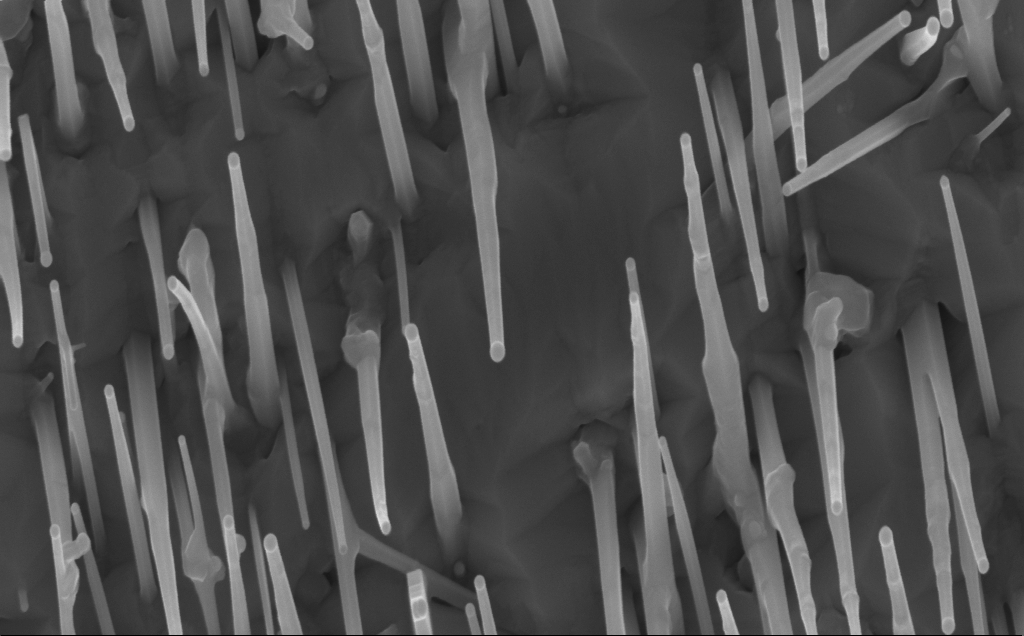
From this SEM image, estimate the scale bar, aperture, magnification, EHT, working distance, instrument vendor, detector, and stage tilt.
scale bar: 200 nm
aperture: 30 µm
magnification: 80 K X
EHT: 10 kV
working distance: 7 mm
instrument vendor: Zeiss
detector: InLens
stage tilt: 0°